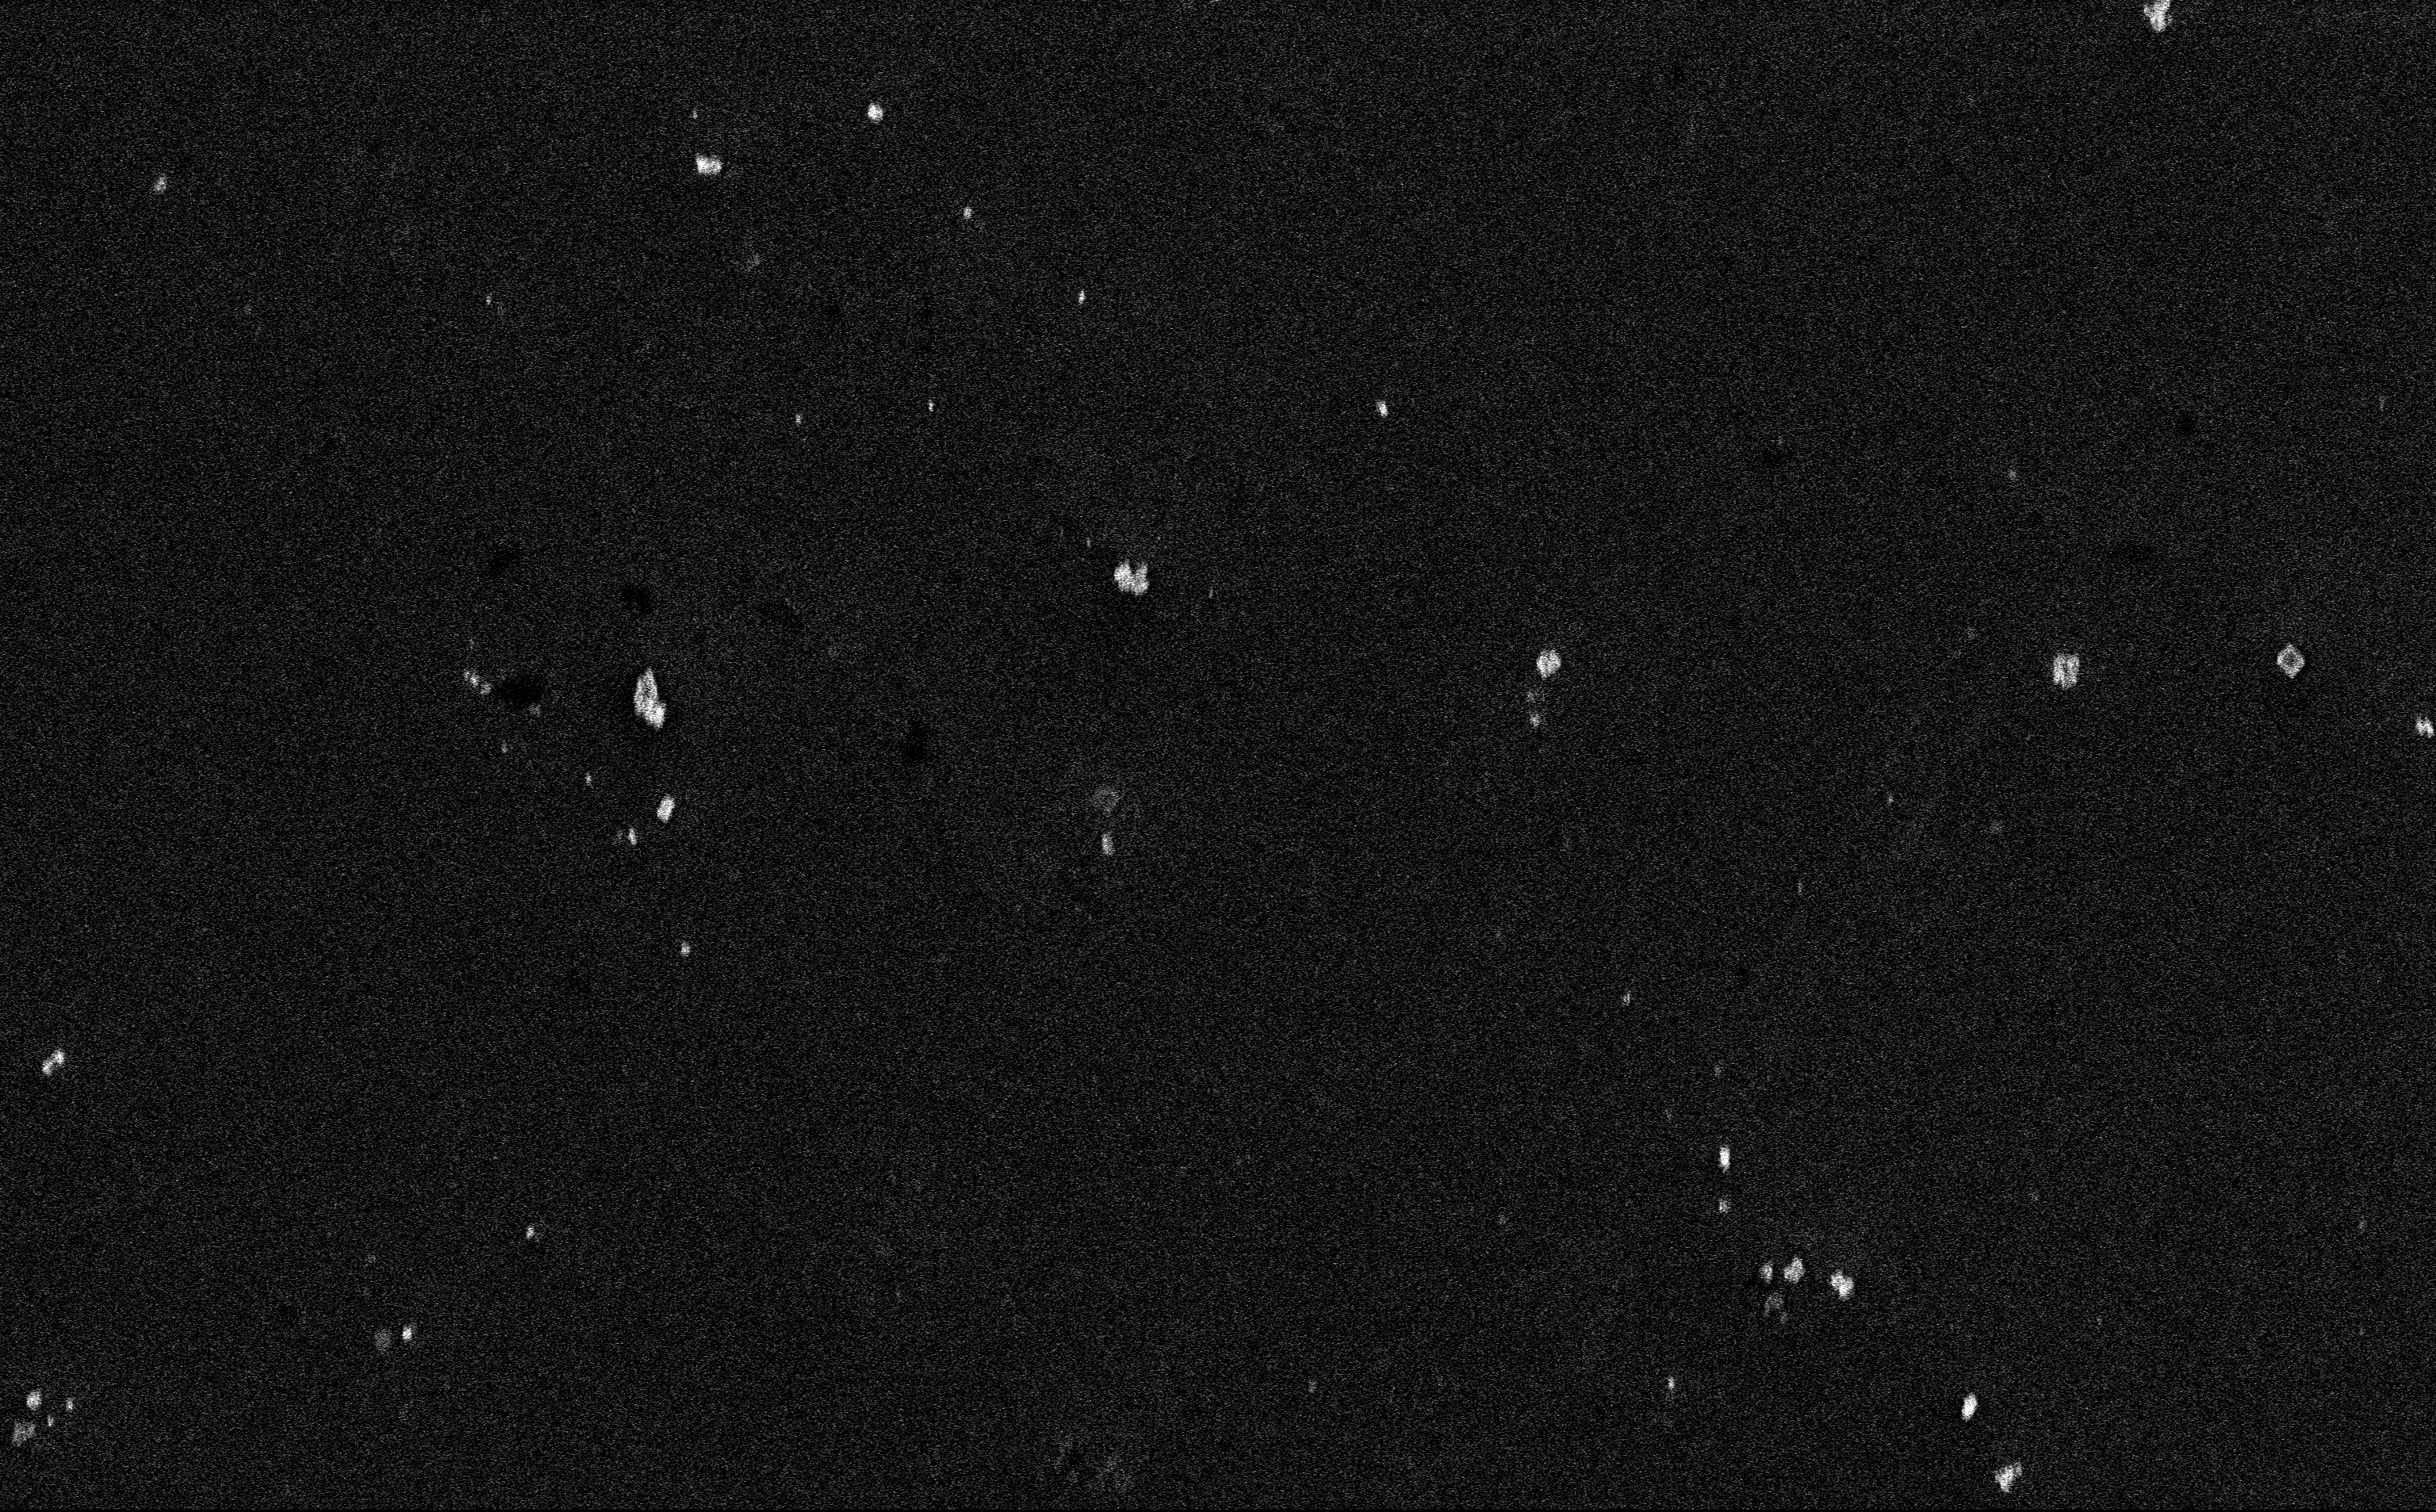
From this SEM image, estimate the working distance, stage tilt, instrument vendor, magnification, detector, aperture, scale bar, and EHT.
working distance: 3 mm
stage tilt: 0°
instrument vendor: Zeiss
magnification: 12.85 K X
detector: InLens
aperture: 30 µm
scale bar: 2000 nm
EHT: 3 kV